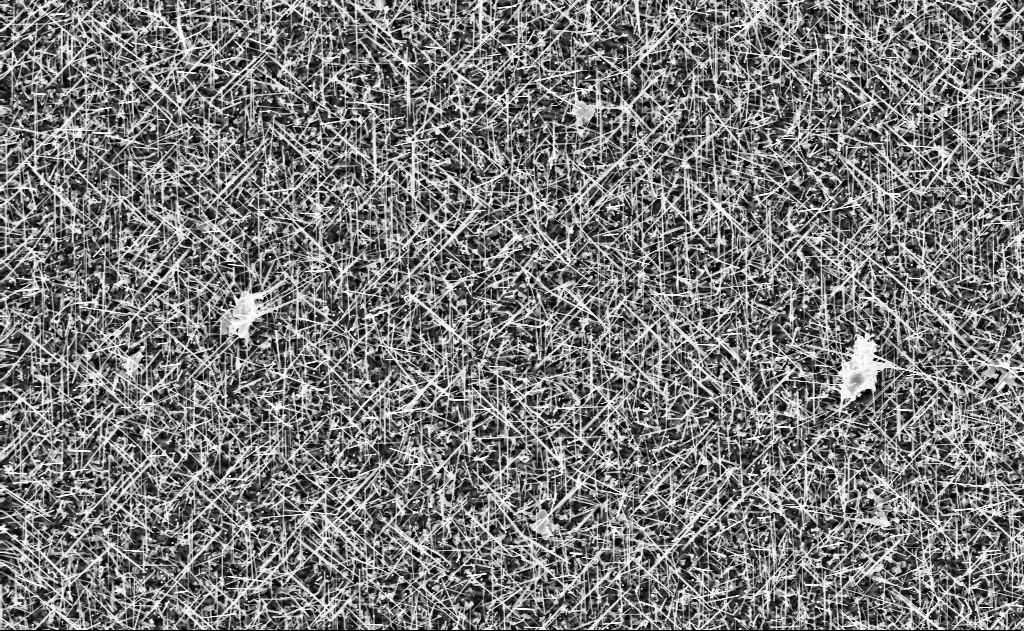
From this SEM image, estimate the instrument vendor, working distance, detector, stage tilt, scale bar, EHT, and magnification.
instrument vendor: Zeiss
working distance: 10 mm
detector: InLens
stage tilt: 0°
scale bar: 2000 nm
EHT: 10 kV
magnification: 10 K X